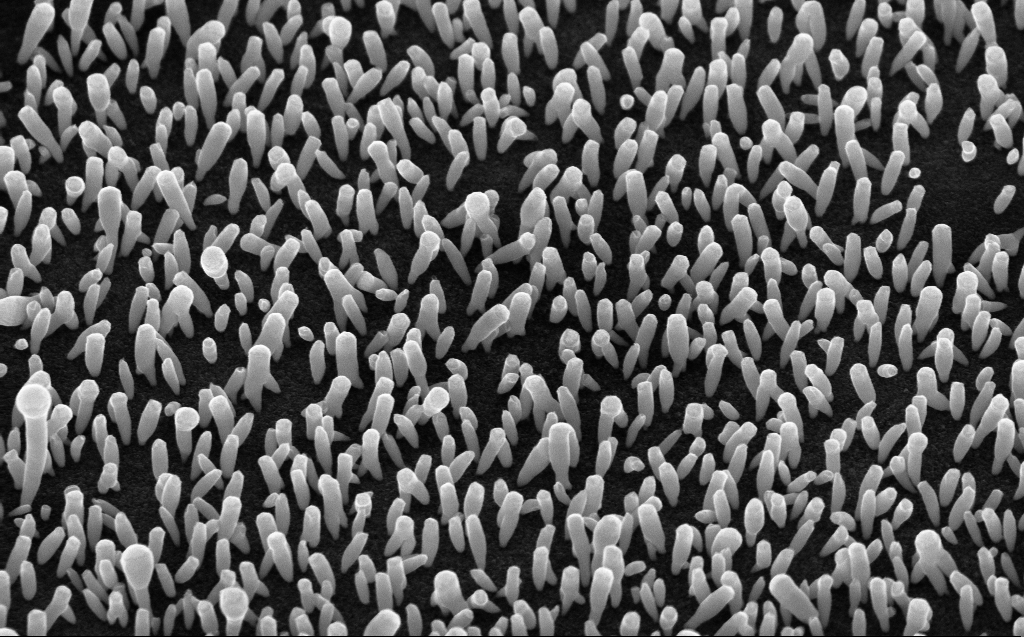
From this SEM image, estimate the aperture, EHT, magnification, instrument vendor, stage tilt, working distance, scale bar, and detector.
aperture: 30 µm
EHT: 10 kV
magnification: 50 K X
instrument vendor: Zeiss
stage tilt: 45°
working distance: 5 mm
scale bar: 1000 nm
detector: InLens